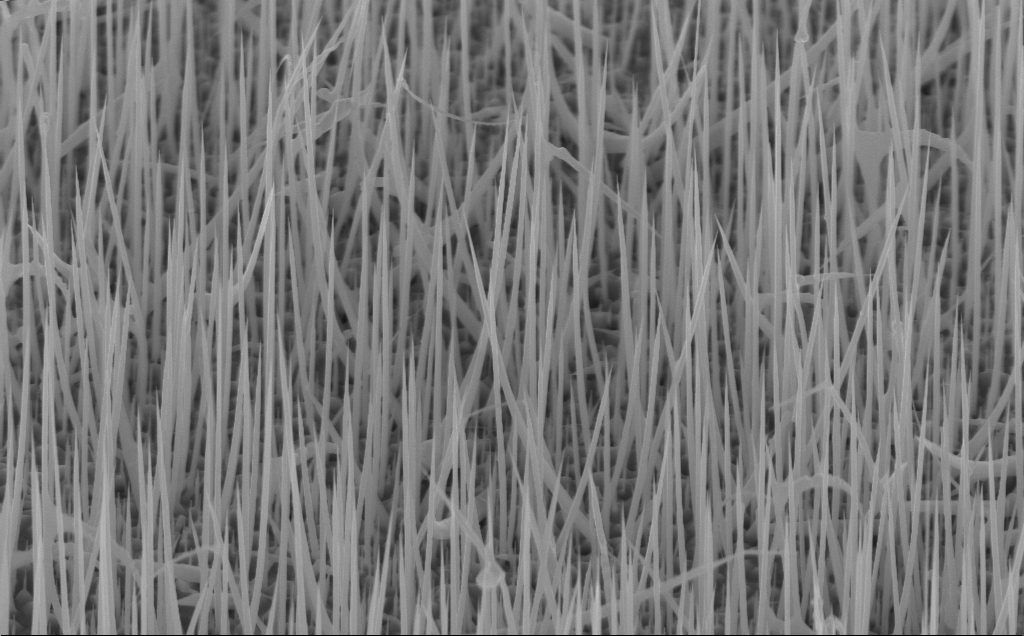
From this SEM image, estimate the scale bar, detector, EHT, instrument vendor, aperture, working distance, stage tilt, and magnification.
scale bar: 1000 nm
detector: InLens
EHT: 10 kV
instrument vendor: Zeiss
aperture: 30 µm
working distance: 7 mm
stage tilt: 45°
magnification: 20 K X